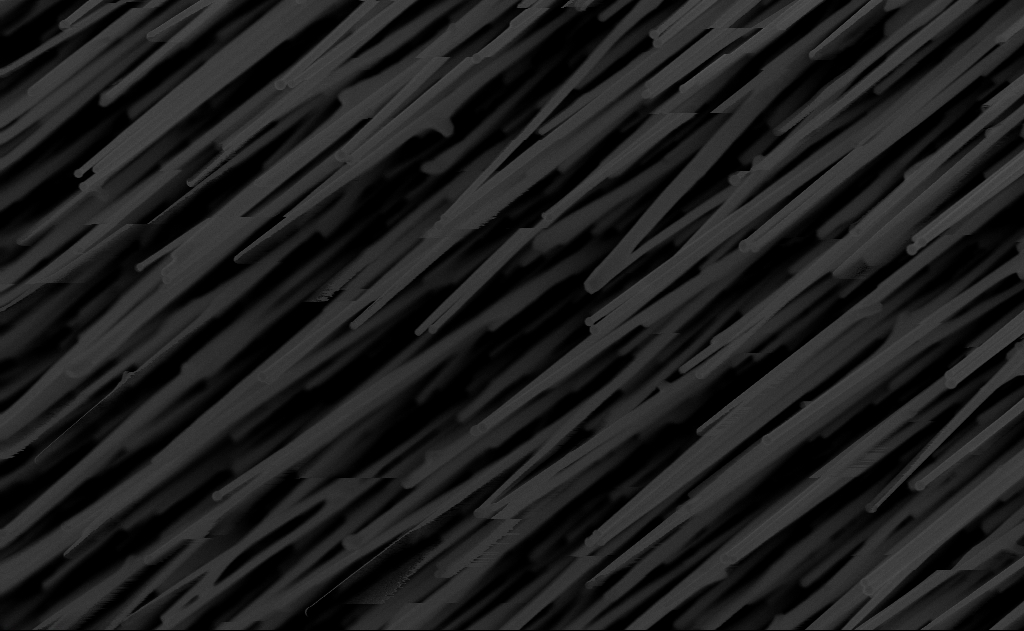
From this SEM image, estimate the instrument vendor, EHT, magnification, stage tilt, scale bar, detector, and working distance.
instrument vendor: Zeiss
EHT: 10 kV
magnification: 40 K X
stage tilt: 0°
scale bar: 1000 nm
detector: InLens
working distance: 10 mm